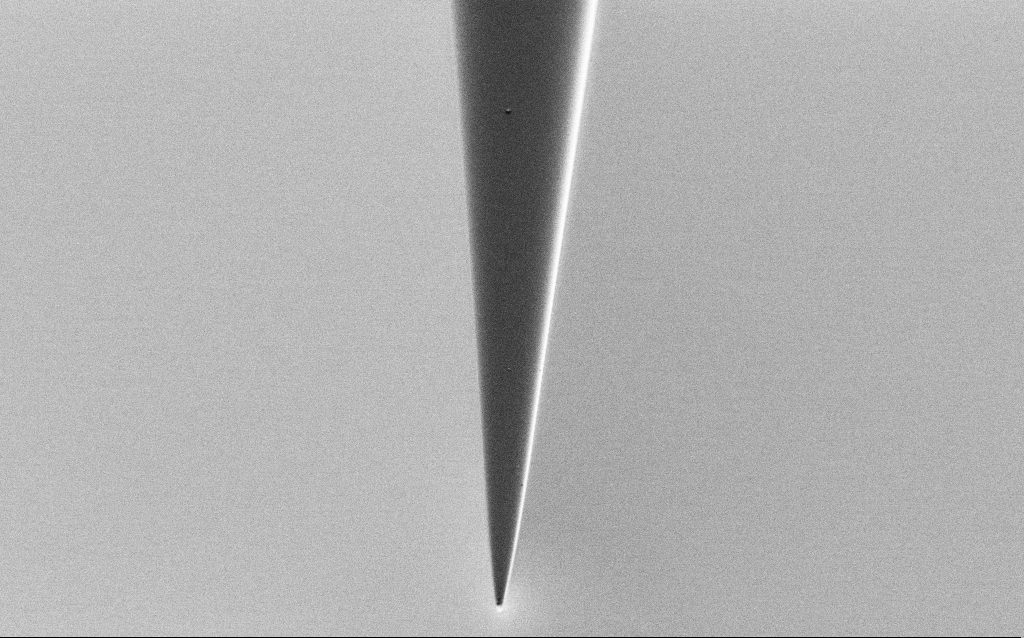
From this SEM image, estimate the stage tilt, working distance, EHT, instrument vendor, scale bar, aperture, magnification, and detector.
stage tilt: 45°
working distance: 6 mm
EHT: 2 kV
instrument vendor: Zeiss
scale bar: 10000 nm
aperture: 30 µm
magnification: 5 K X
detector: SE2